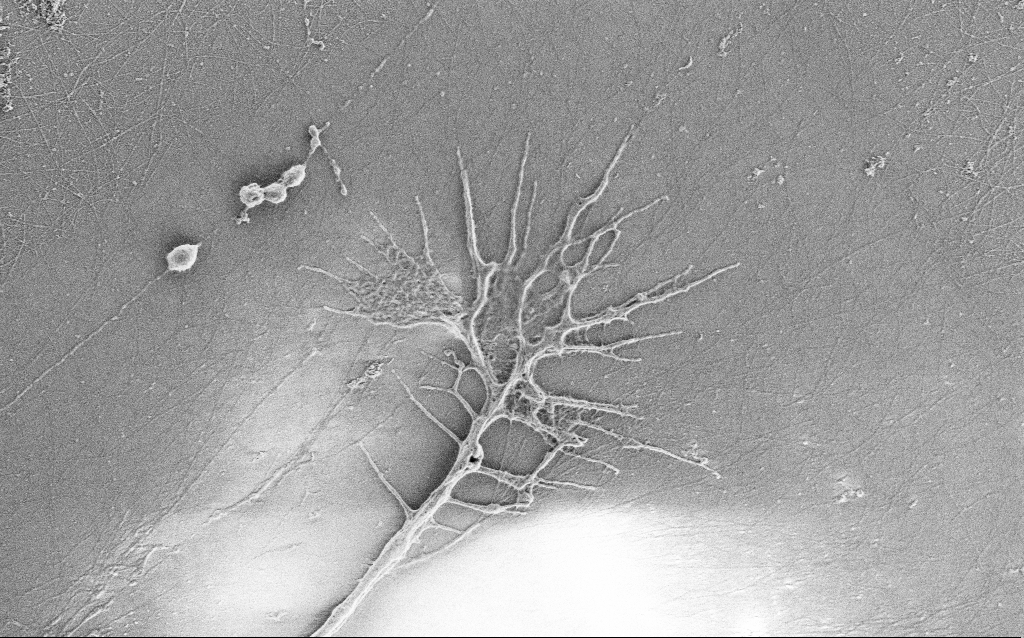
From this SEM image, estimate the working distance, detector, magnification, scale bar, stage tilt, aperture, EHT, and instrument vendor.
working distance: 4 mm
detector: SE2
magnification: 10 K X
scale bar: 2000 nm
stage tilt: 0°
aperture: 30 µm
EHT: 1 kV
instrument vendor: Zeiss